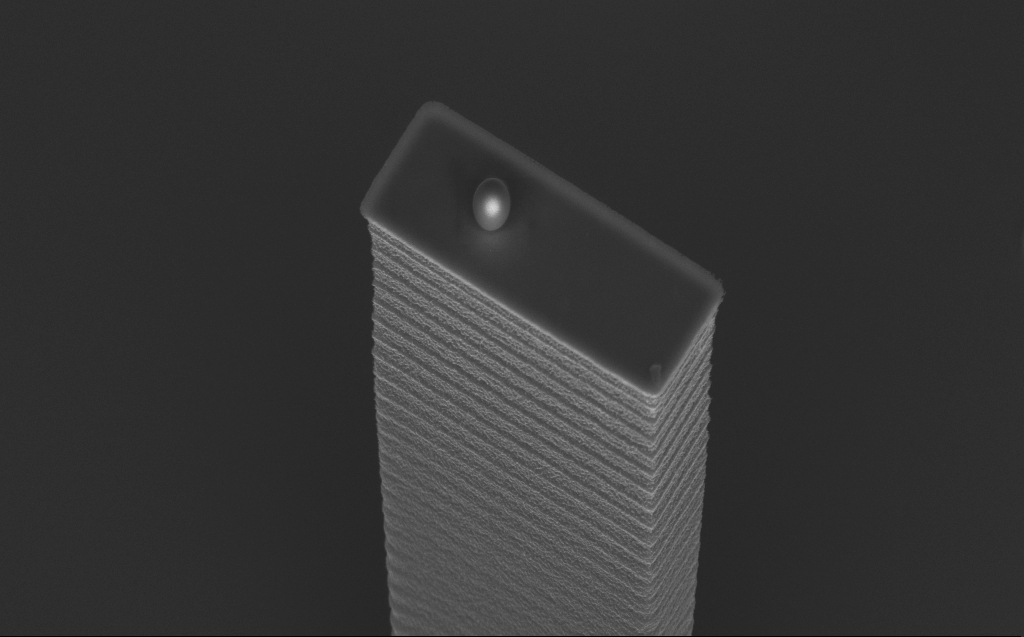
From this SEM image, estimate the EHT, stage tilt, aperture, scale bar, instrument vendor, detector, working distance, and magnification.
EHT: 5 kV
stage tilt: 45°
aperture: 30 µm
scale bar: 1000 nm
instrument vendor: Zeiss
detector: InLens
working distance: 7 mm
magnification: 17.17 K X